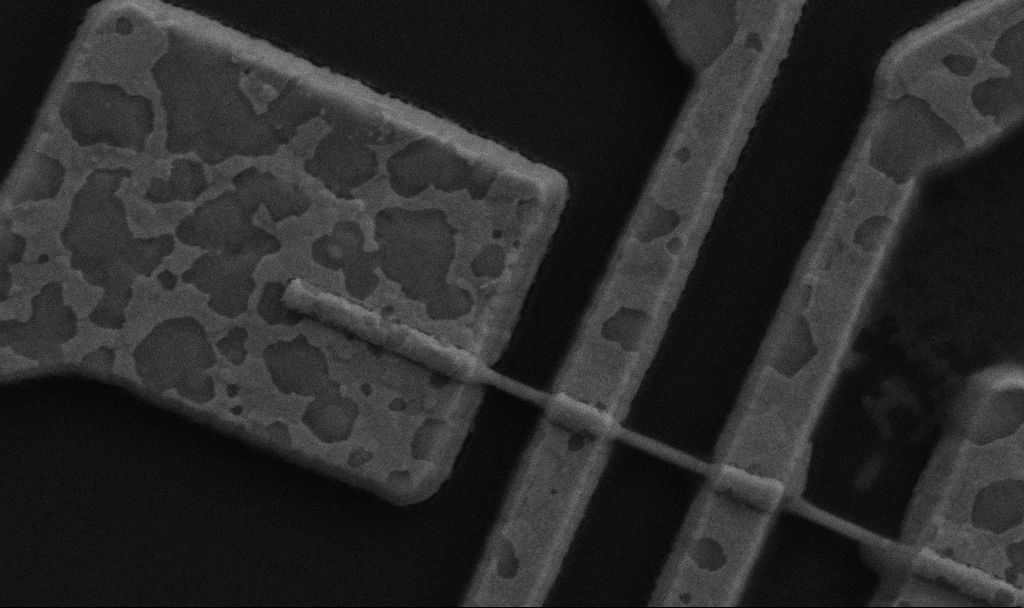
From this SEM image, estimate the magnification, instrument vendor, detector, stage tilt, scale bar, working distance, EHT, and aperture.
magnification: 60 K X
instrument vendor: Zeiss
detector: SE2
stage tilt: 0°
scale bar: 1000 nm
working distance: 8.7 mm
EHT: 5 kV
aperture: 30 µm